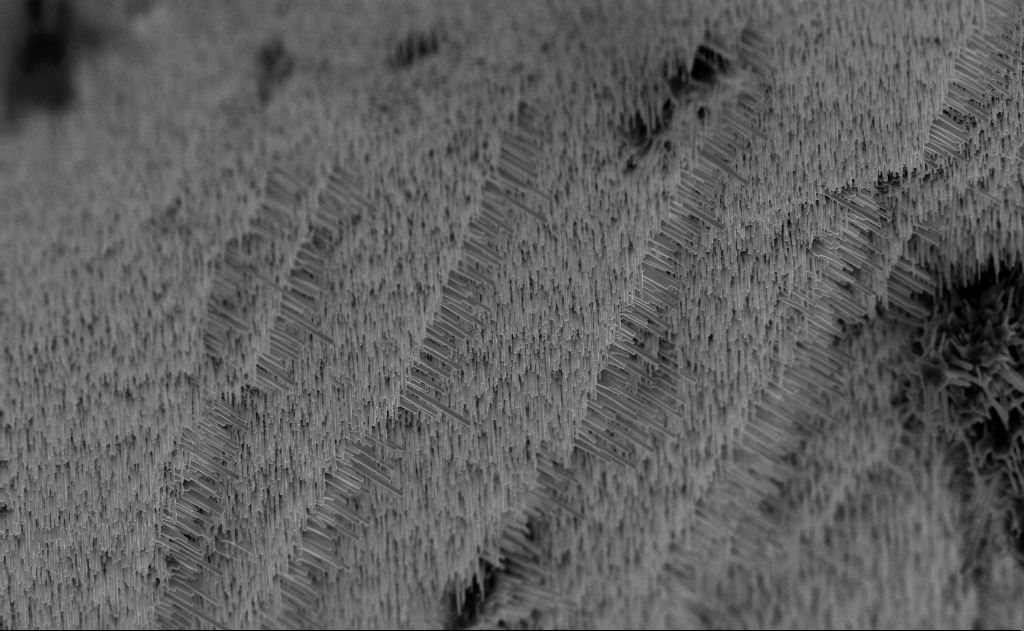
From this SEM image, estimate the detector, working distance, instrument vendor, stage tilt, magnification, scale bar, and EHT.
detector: InLens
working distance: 6 mm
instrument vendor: Zeiss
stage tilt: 0°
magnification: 10 K X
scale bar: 2000 nm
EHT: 10 kV